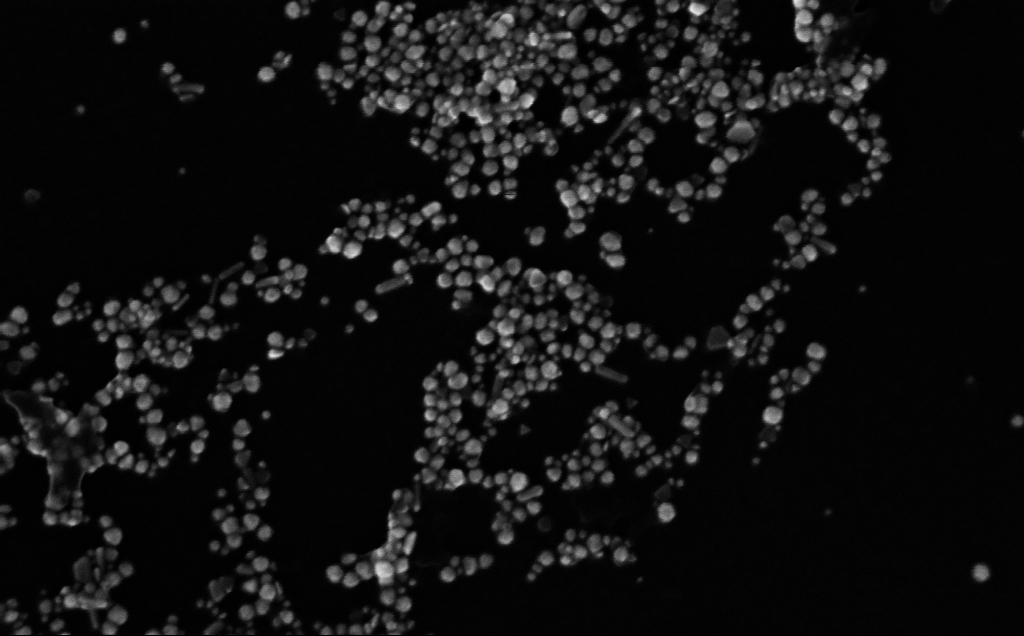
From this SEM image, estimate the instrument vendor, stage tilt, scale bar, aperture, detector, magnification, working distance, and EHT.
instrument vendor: Zeiss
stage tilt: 0°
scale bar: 200 nm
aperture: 30 µm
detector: InLens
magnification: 233.89 K X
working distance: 4 mm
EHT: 10 kV